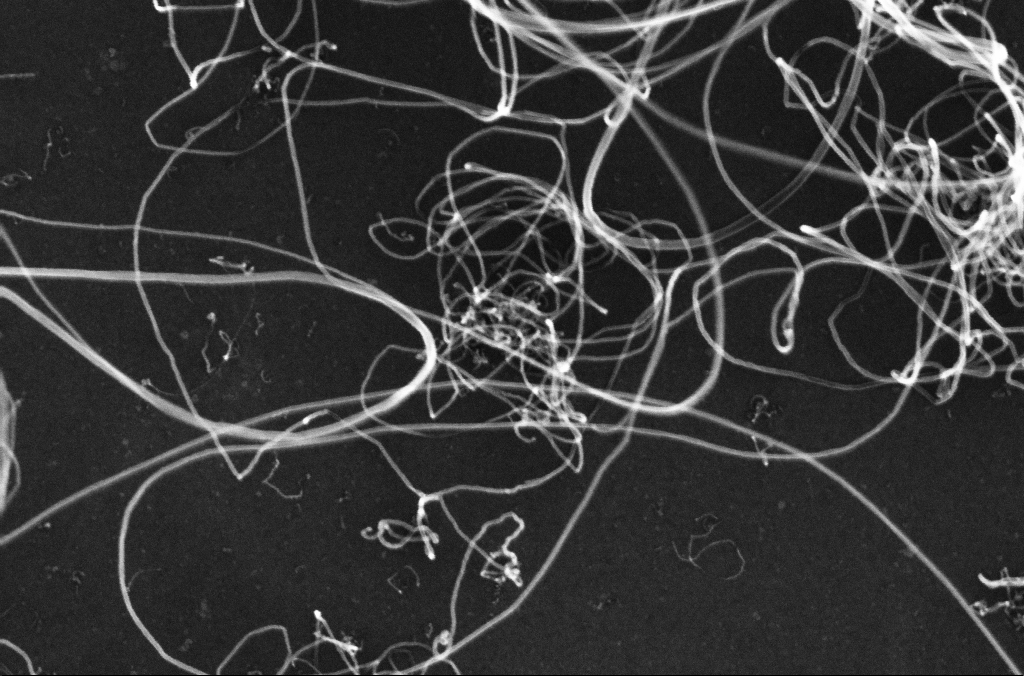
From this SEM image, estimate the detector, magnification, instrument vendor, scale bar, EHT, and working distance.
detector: InLens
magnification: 150 K X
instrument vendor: Zeiss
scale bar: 100 nm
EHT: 10 kV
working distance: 3.3 mm